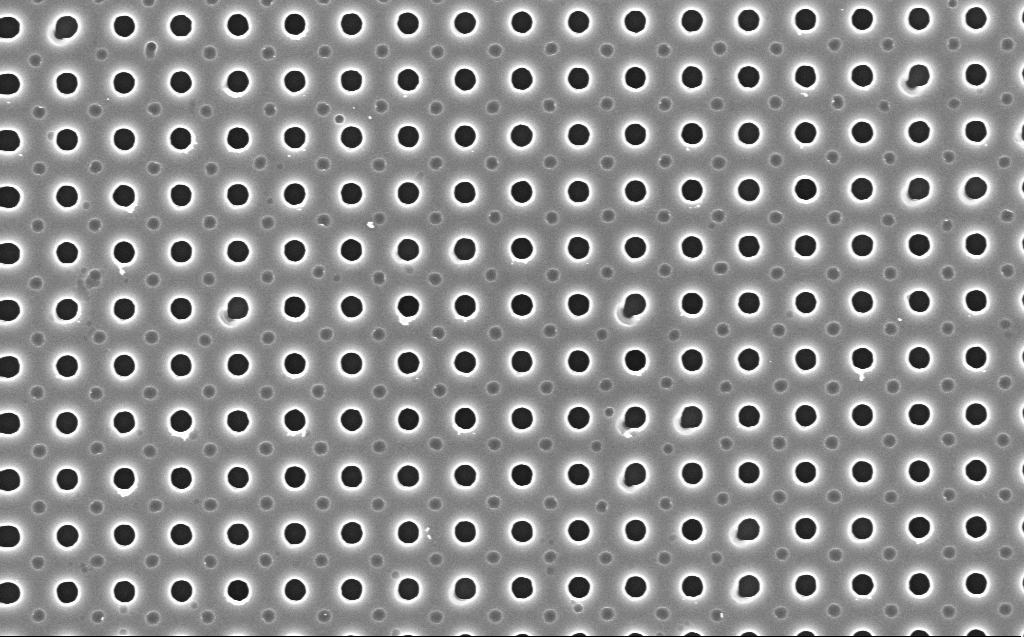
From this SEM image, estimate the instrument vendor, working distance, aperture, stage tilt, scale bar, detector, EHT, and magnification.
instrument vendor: Zeiss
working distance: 4 mm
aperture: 30 µm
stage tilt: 0°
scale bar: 1000 nm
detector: InLens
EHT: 5 kV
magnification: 20 K X